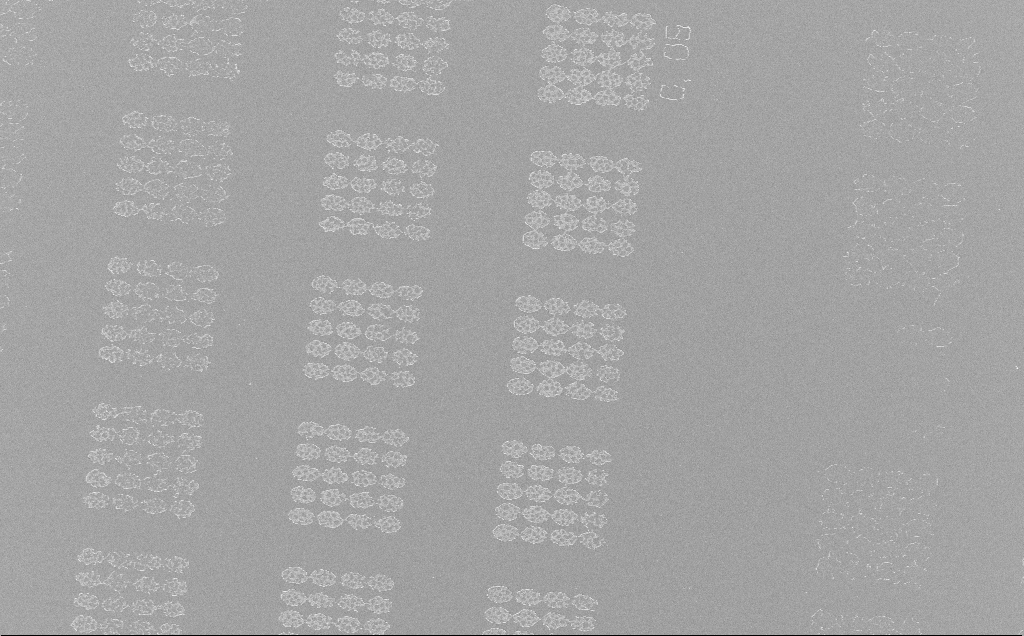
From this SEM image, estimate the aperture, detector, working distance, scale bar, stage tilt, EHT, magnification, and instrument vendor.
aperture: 30 µm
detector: InLens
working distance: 6 mm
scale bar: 20000 nm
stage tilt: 0°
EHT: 5 kV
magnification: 1.08 K X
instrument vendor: Zeiss